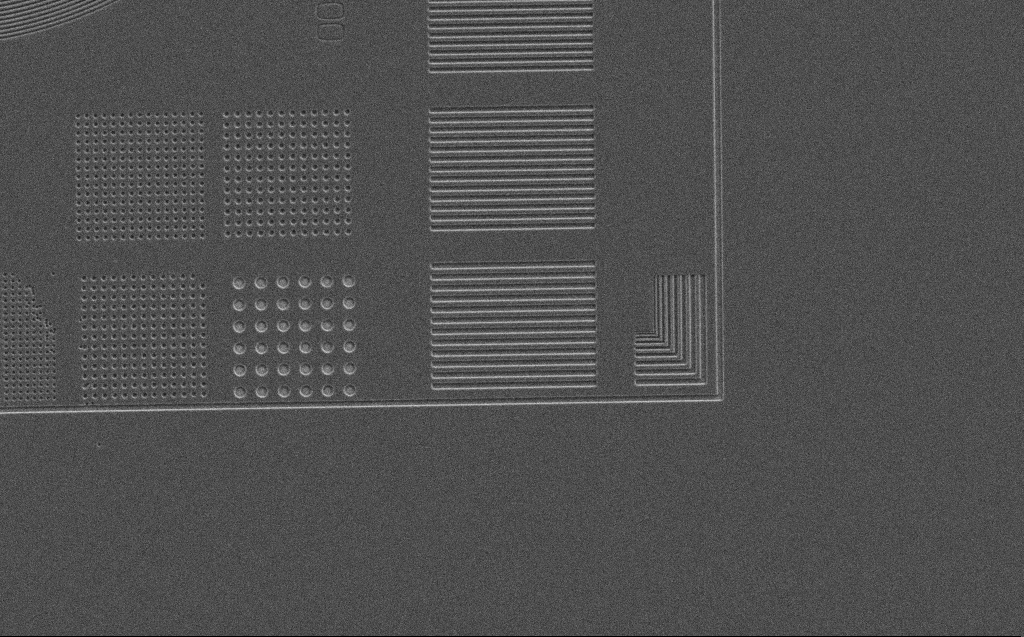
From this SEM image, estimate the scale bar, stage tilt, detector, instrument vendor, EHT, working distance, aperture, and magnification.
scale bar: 20000 nm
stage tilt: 0°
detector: SE2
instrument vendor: Zeiss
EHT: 4 kV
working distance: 7 mm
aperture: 30 µm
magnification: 2.03 K X